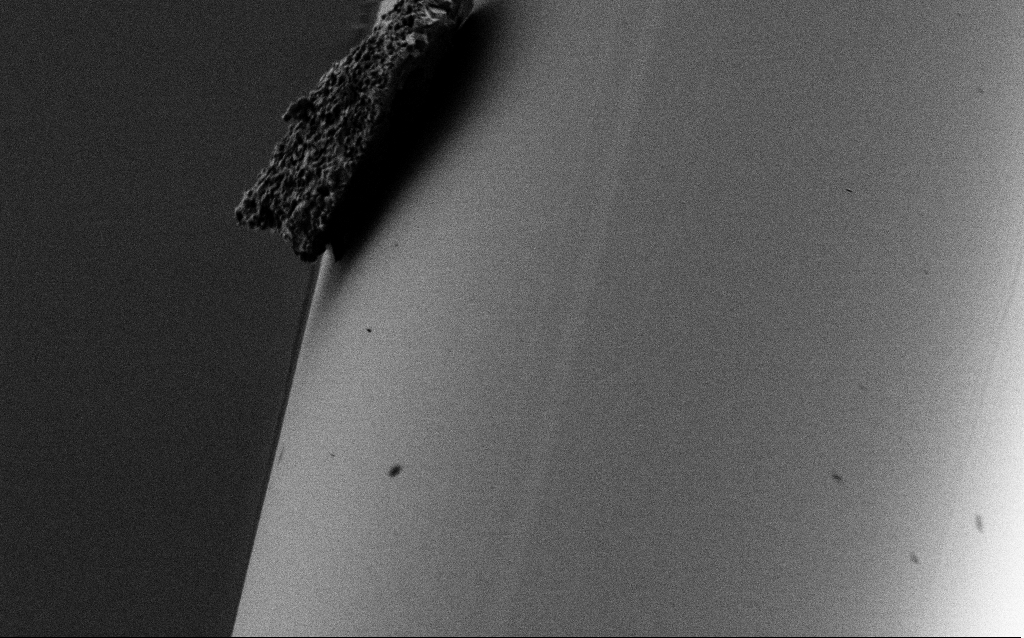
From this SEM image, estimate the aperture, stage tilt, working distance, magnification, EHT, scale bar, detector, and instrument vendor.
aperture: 30 µm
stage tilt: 45°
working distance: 6.6 mm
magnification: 10 K X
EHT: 1 kV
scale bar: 2000 nm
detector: SE2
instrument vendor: Zeiss